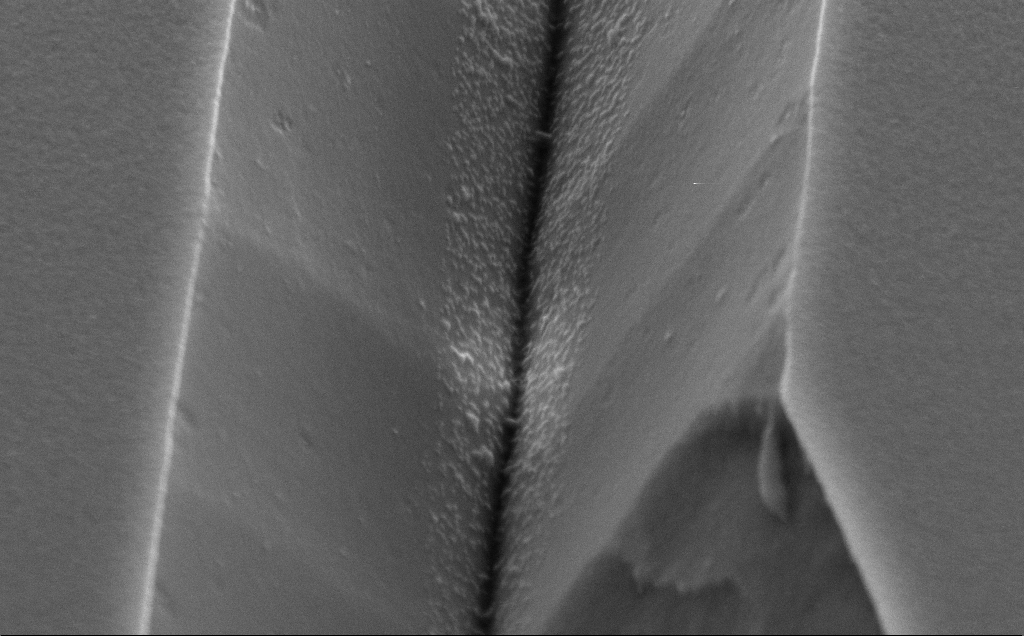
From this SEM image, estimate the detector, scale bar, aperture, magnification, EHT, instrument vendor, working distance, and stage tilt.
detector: SE2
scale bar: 200 nm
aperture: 30 µm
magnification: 73.99 K X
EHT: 5 kV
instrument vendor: Zeiss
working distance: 10 mm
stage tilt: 50°